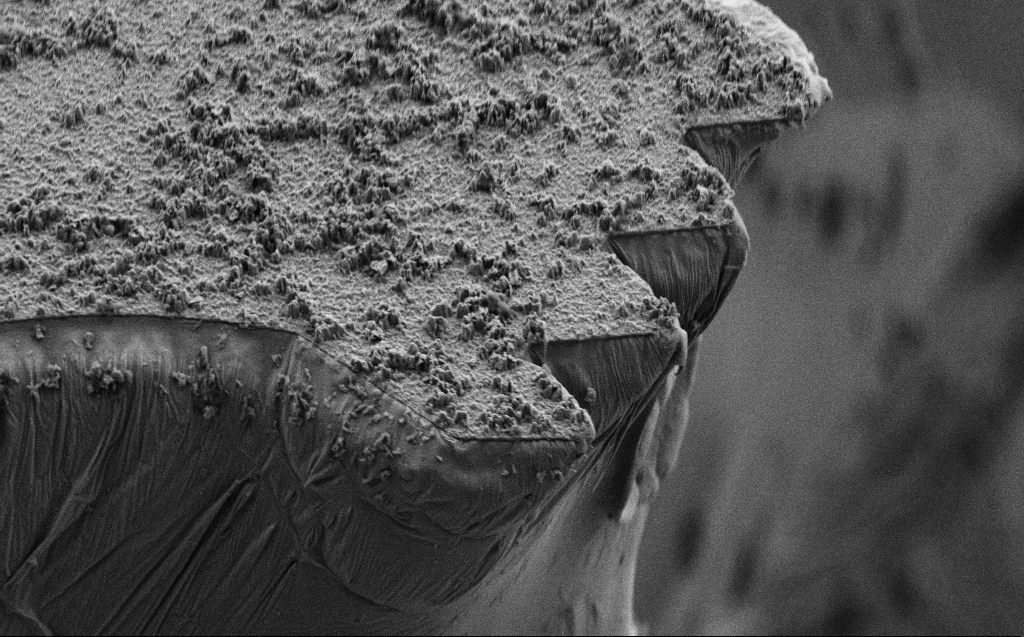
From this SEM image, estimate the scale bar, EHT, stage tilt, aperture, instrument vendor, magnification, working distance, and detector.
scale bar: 10000 nm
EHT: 5 kV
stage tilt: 45°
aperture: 30 µm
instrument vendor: Zeiss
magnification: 4.44 K X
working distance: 8 mm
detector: SE2